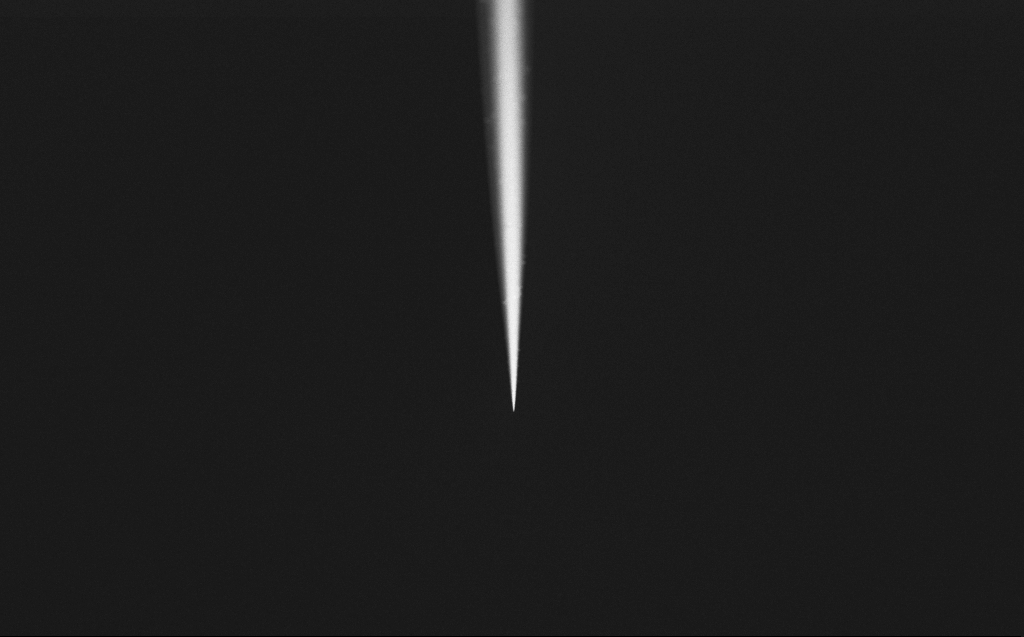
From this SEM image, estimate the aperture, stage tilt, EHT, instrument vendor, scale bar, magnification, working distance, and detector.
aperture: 30 µm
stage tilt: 45°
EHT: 2 kV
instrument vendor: Zeiss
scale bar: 20000 nm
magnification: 1 K X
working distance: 4 mm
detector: InLens